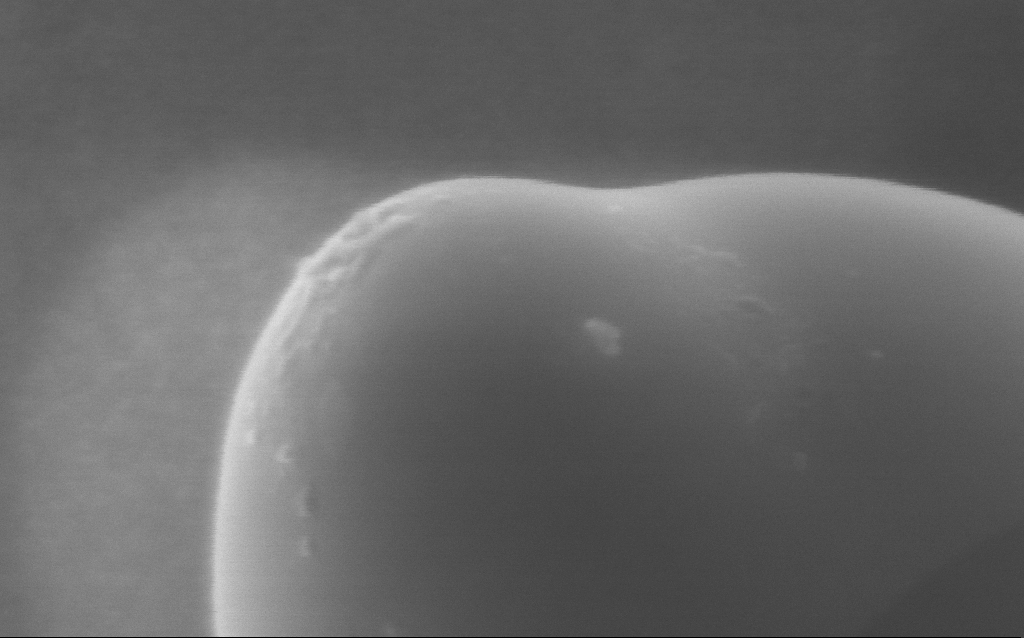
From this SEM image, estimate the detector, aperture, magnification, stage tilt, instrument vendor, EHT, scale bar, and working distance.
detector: InLens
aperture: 30 µm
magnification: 270 K X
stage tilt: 0°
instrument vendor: Zeiss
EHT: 5 kV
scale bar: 100 nm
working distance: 4 mm